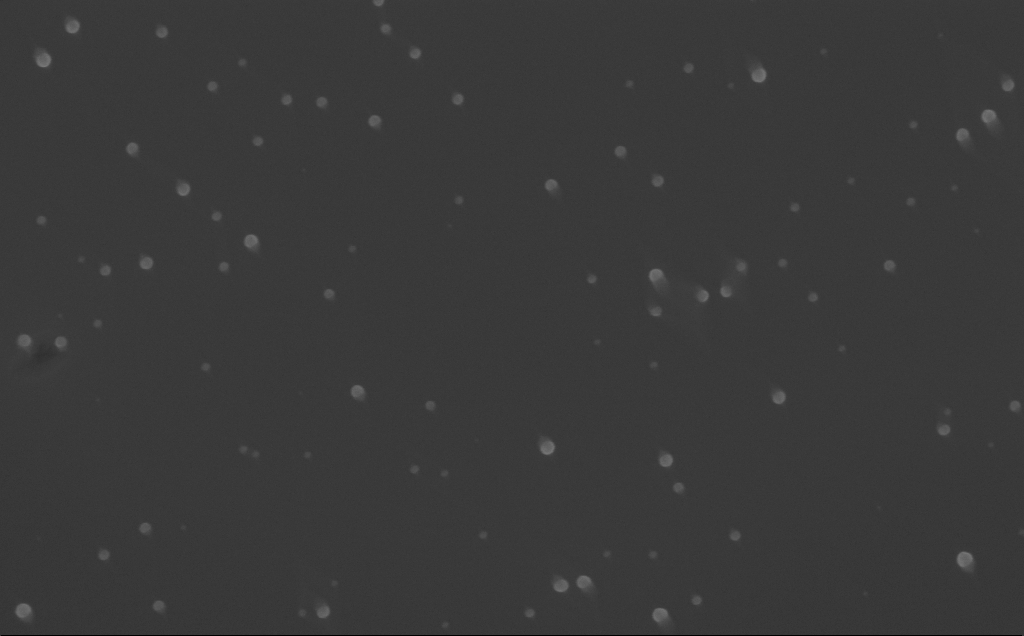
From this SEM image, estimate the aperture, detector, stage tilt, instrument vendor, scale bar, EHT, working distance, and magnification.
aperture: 30 µm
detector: InLens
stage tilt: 0°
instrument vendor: Zeiss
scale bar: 200 nm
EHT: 10 kV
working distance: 6 mm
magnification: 80 K X